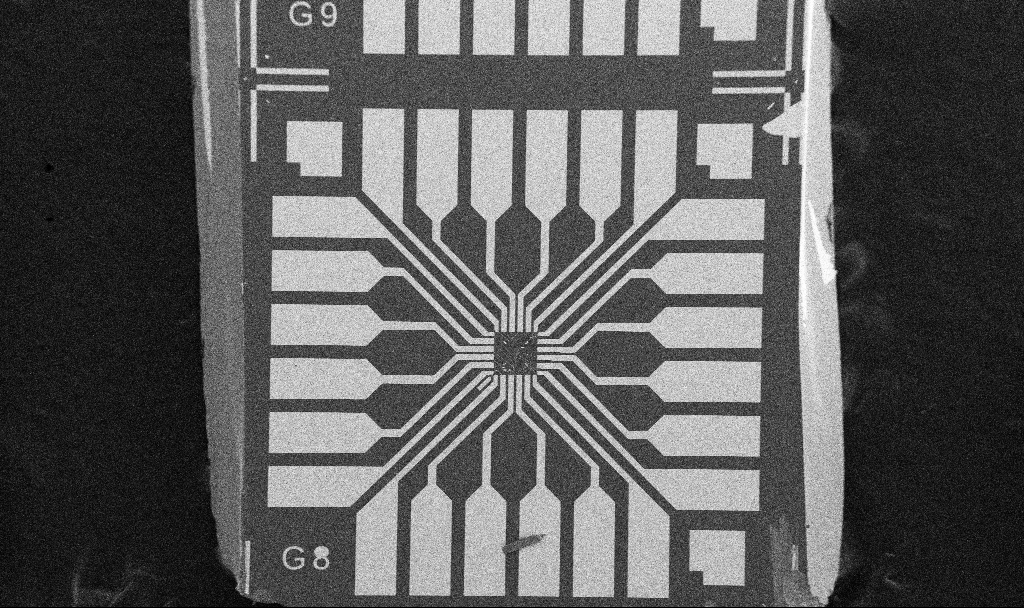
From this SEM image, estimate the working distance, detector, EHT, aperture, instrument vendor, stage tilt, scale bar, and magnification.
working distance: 10.7 mm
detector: SE2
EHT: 5 kV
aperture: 30 µm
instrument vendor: Zeiss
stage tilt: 0°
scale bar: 200000 nm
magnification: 0.1 K X